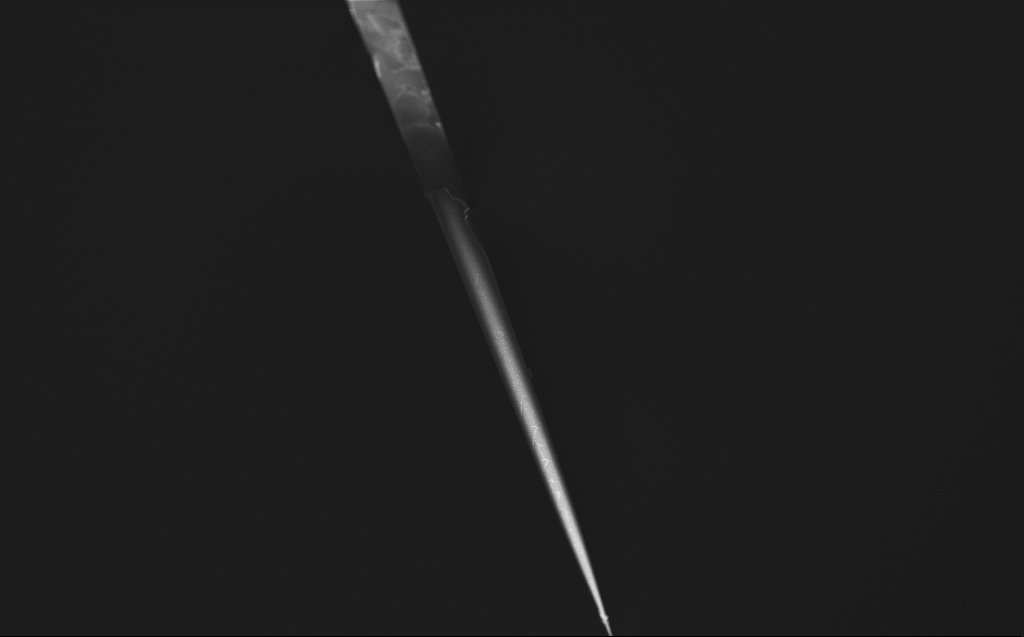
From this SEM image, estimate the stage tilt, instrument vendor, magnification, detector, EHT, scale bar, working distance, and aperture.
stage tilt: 45°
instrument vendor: Zeiss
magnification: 0.5 K X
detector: InLens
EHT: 1 kV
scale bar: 100000 nm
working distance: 4 mm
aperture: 30 µm